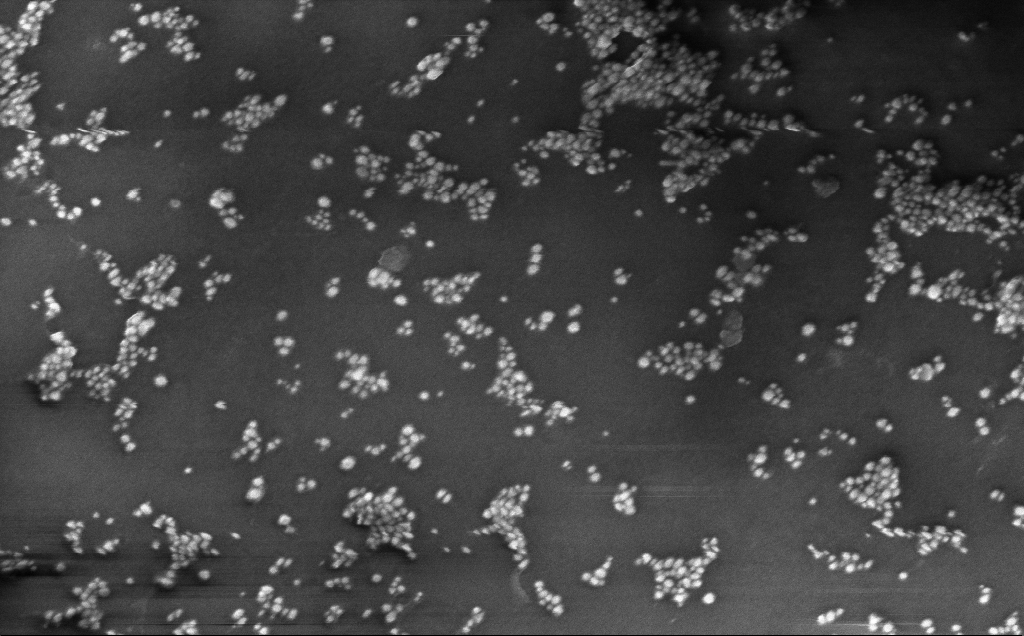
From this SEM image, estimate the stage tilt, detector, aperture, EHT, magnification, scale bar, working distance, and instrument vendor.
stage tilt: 0°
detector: InLens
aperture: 30 µm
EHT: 10 kV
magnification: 144.66 K X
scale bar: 200 nm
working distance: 4 mm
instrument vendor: Zeiss